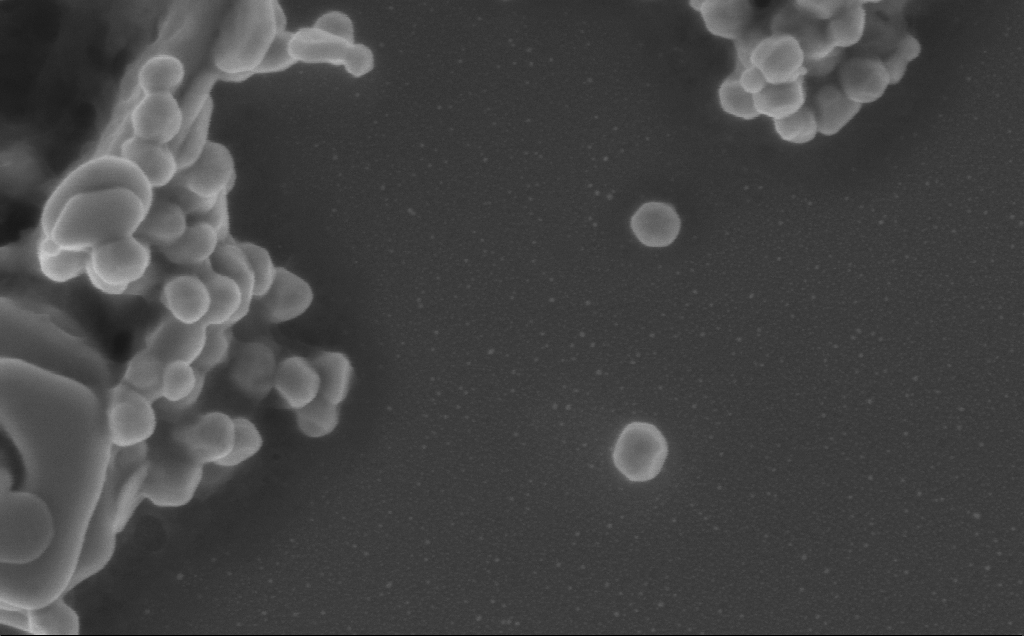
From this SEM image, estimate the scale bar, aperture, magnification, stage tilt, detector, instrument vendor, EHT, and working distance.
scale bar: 200 nm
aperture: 30 µm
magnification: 132.51 K X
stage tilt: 0°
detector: InLens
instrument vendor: Zeiss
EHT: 5 kV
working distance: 3 mm